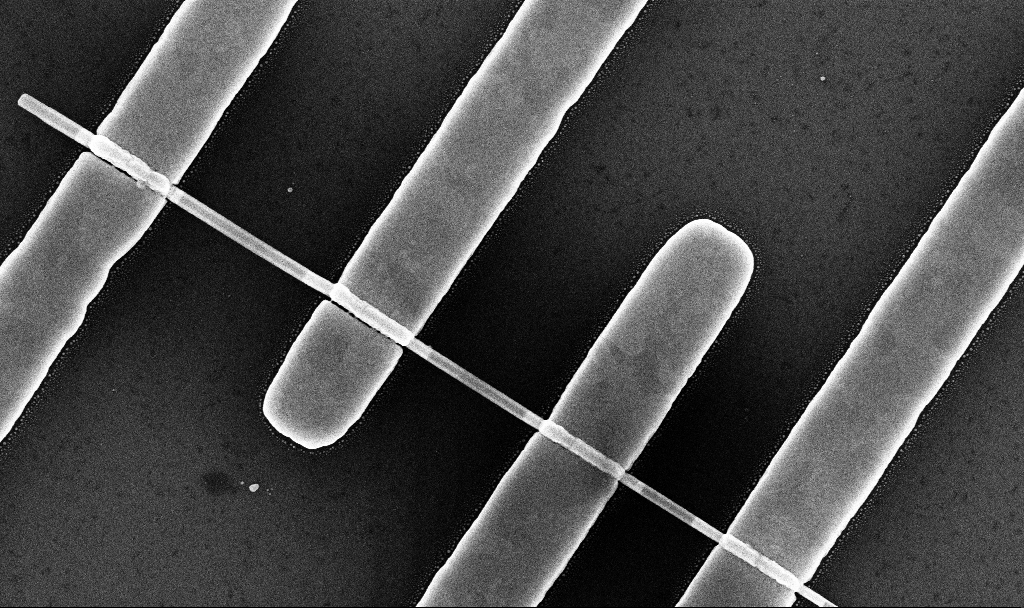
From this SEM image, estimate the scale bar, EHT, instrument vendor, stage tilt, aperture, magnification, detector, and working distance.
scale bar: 1000 nm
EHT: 10 kV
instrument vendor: Zeiss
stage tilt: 0°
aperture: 30 µm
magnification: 56.42 K X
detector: InLens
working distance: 7.8 mm